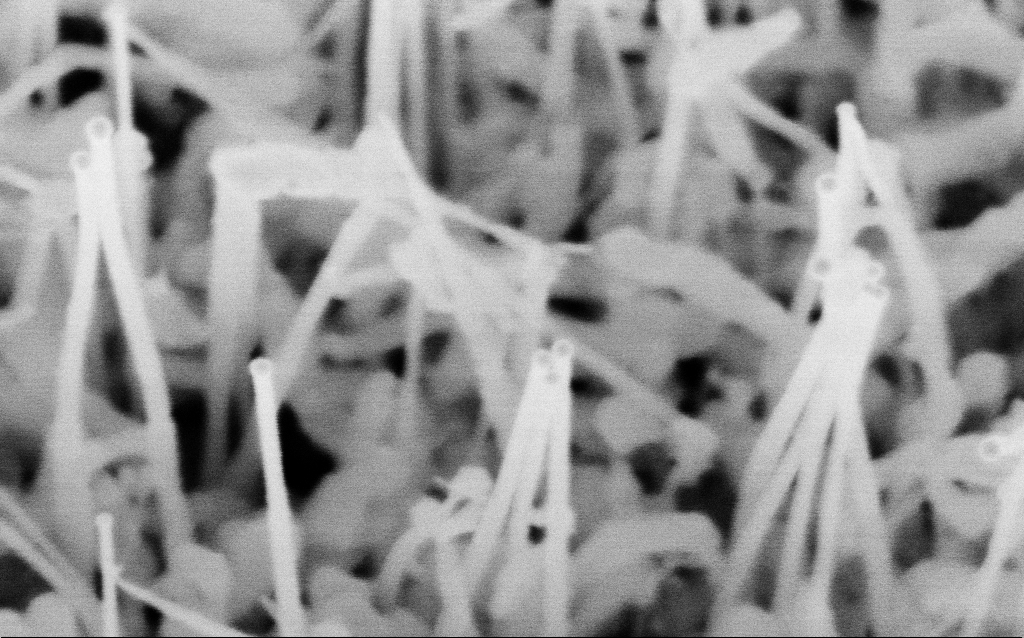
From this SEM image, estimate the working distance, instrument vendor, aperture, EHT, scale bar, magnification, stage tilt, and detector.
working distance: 7.3 mm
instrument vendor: Zeiss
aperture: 30 µm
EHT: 10 kV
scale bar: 100 nm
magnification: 243.35 K X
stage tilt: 35°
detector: InLens